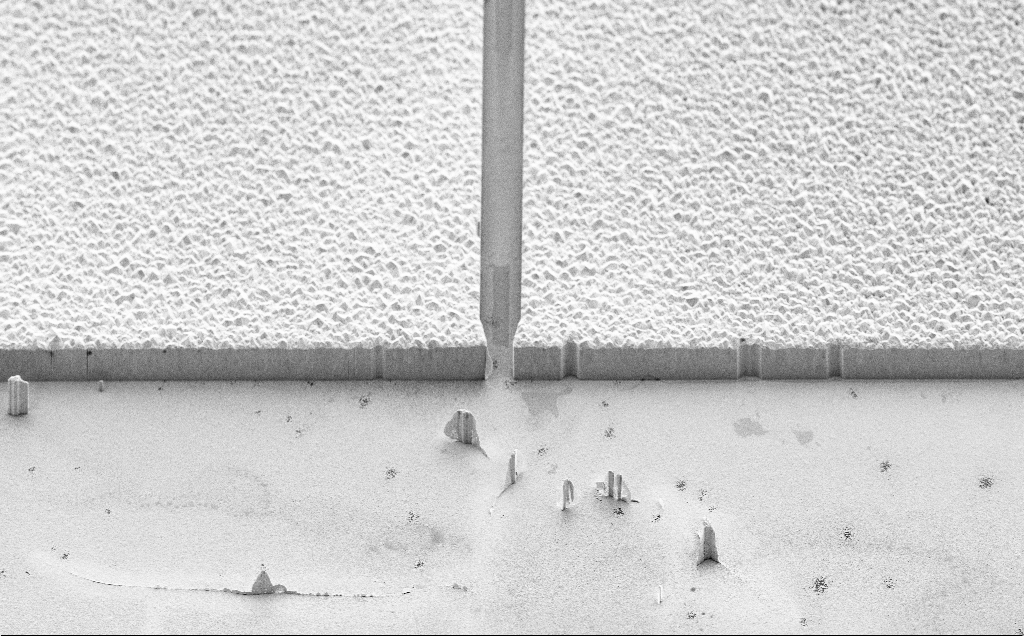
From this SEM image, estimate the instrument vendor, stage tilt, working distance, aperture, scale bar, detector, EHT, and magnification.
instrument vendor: Zeiss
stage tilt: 45°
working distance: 9 mm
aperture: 30 µm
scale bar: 20000 nm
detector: SE2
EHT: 5 kV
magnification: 1.49 K X